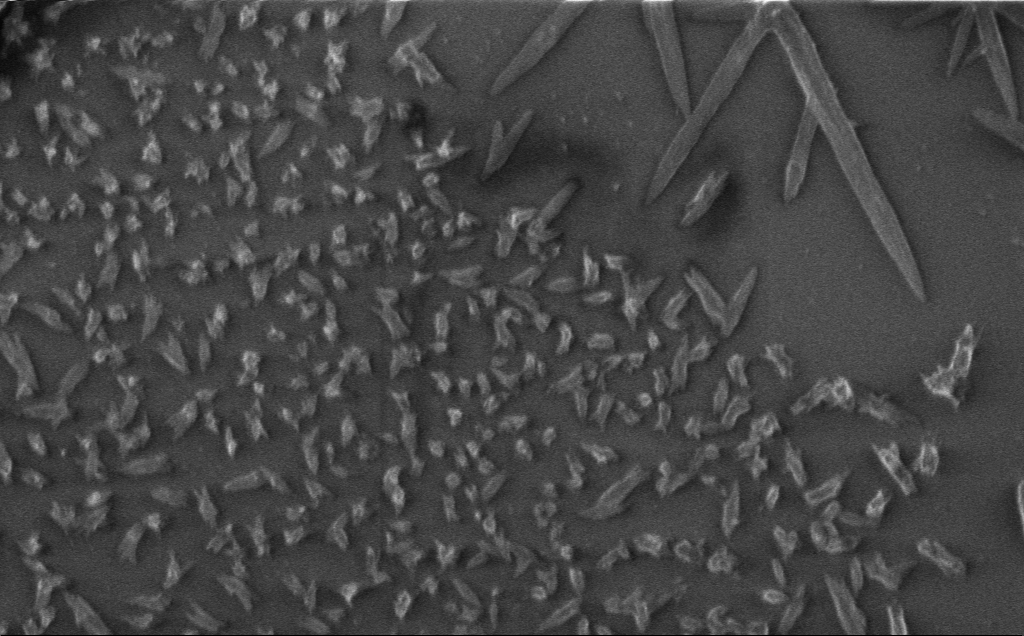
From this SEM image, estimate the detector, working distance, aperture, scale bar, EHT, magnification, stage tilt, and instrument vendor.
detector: InLens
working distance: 3 mm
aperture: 30 µm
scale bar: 2000 nm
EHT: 1 kV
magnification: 13.6 K X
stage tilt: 0°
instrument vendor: Zeiss